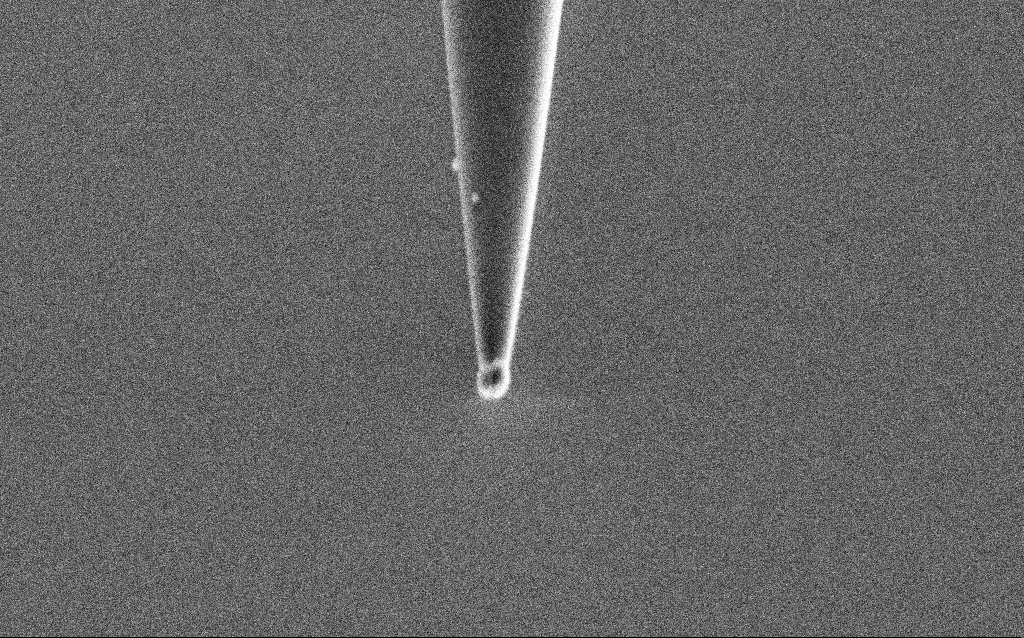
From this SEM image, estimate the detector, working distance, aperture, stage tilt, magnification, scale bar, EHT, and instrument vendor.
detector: SE2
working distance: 6.5 mm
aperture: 30 µm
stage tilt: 44.9°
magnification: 50 K X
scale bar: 1000 nm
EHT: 2 kV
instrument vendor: Zeiss